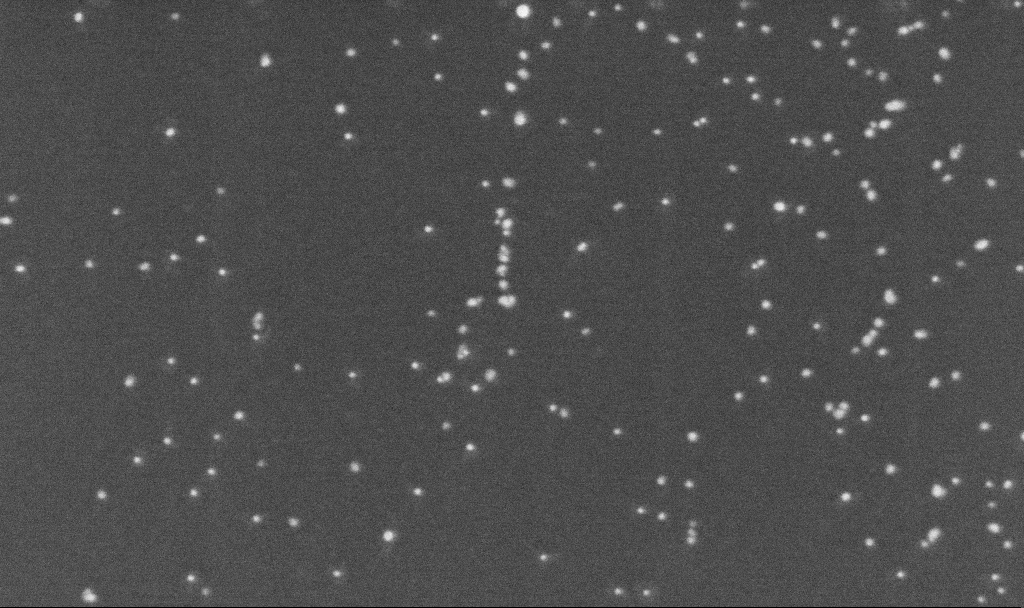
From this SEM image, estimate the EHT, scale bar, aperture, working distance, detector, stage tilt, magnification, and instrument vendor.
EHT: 5 kV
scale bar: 200 nm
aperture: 30 µm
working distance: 3.2 mm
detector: InLens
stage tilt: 0°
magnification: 200 K X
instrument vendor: Zeiss